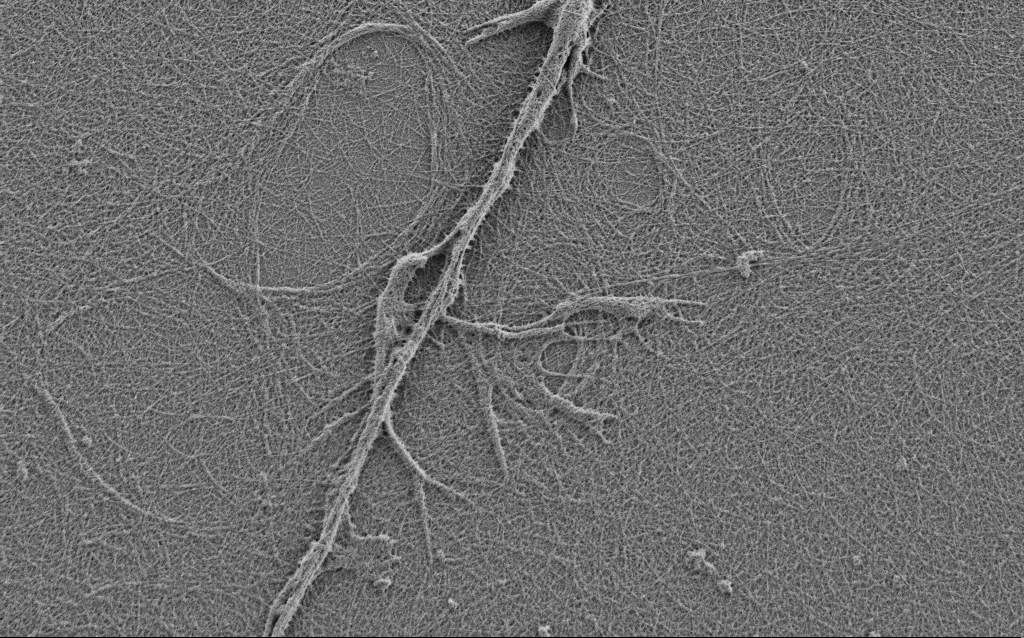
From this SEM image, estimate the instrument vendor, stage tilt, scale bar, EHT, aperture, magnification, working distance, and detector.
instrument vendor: Zeiss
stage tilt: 0°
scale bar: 2000 nm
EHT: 0.9 kV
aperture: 30 µm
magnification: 10 K X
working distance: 4 mm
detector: SE2